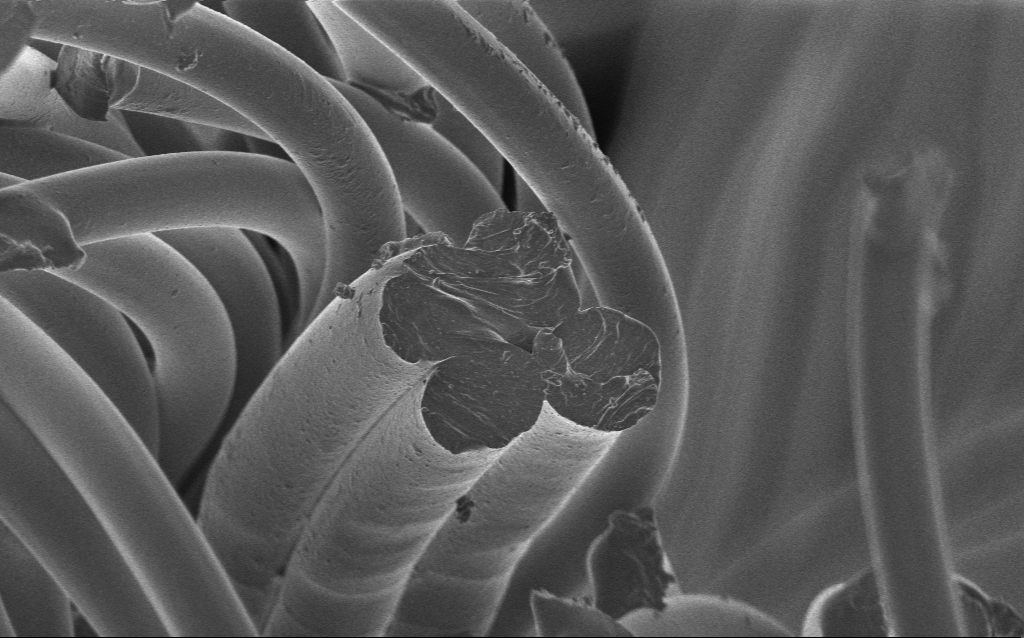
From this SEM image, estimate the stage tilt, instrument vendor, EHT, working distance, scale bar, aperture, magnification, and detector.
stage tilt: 0°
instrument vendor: Zeiss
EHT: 1 kV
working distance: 3 mm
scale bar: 20000 nm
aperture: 30 µm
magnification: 1.23 K X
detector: InLens